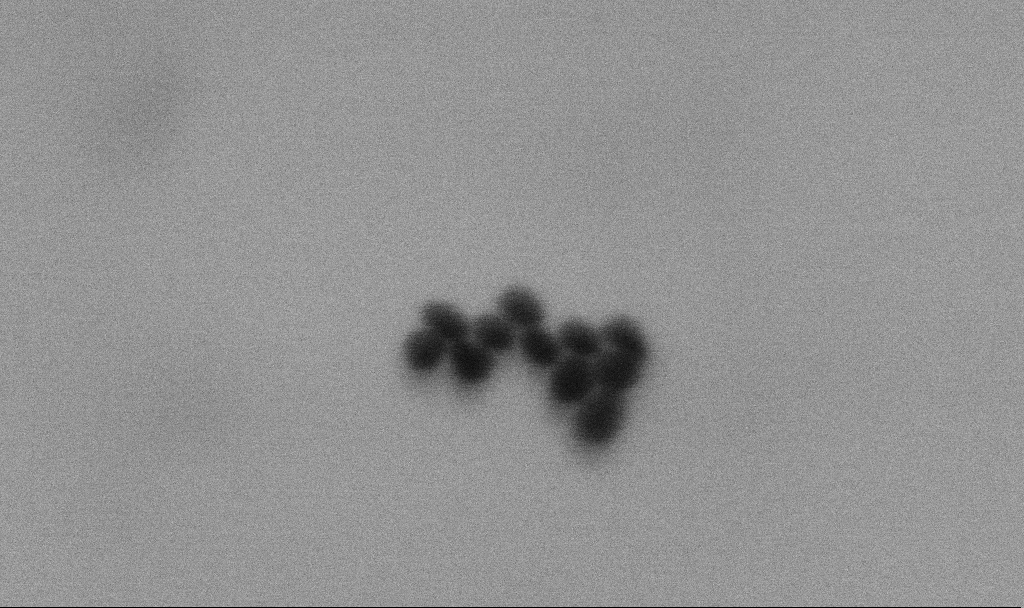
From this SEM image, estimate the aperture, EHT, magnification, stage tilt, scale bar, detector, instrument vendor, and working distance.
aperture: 30 µm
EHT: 4 kV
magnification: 833.97 K X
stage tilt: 0°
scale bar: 20 nm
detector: SE2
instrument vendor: Zeiss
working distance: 4 mm